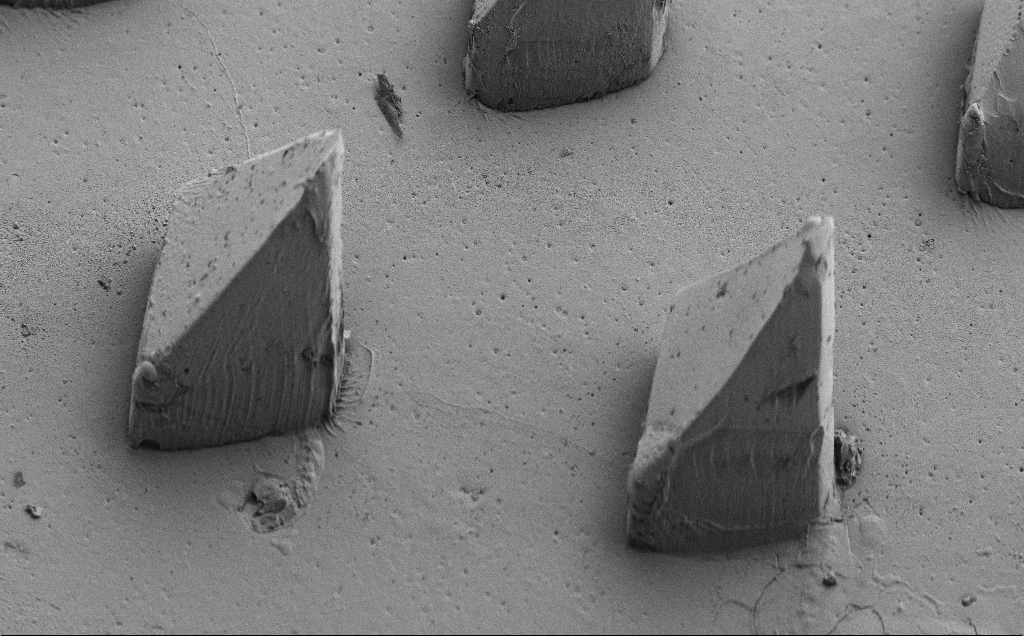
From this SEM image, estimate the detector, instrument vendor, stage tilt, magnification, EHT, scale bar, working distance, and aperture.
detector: SE2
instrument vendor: Zeiss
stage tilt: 39°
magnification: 0.193 K X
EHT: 5 kV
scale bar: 200000 nm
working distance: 8 mm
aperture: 30 µm